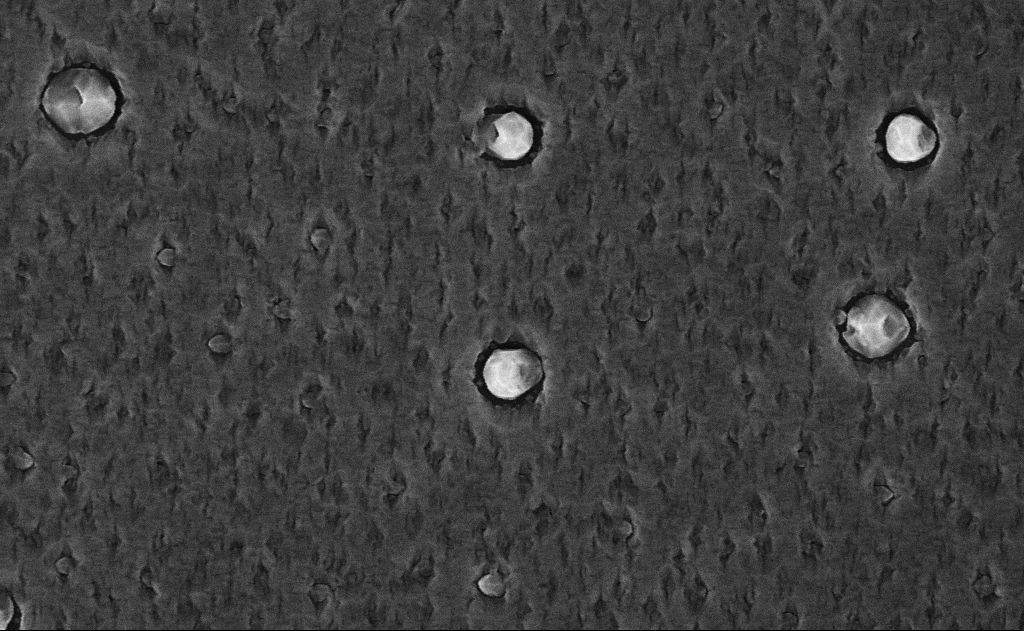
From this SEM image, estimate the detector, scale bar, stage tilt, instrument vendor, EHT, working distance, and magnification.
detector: InLens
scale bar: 1000 nm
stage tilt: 0°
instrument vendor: Zeiss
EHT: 10 kV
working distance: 18 mm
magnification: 40 K X